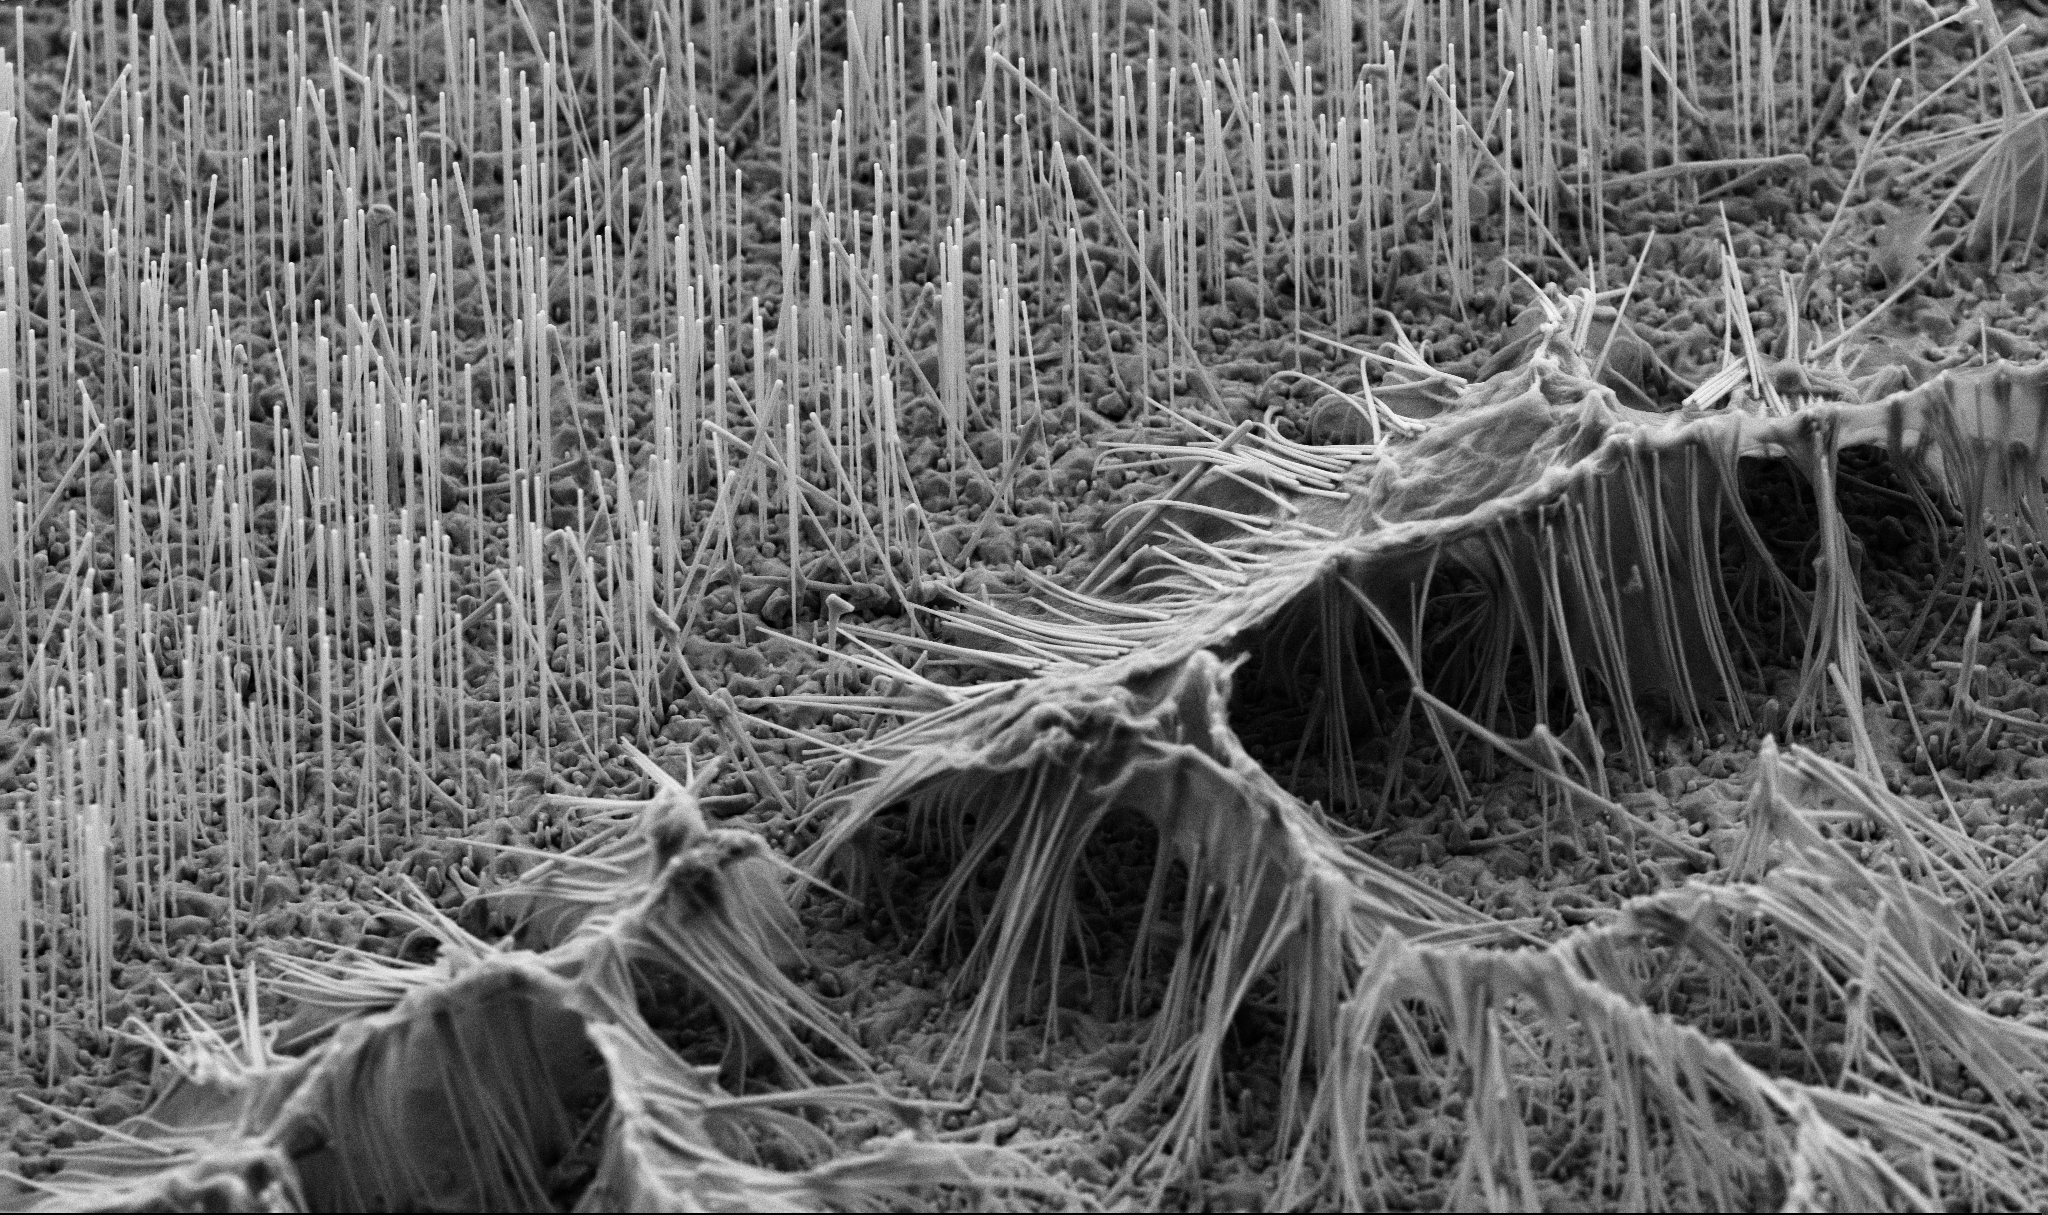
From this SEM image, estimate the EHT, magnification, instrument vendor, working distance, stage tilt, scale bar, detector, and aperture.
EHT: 5 kV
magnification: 8.04 K X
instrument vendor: Zeiss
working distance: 7.2 mm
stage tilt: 45°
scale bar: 2000 nm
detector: SE2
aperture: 30 µm